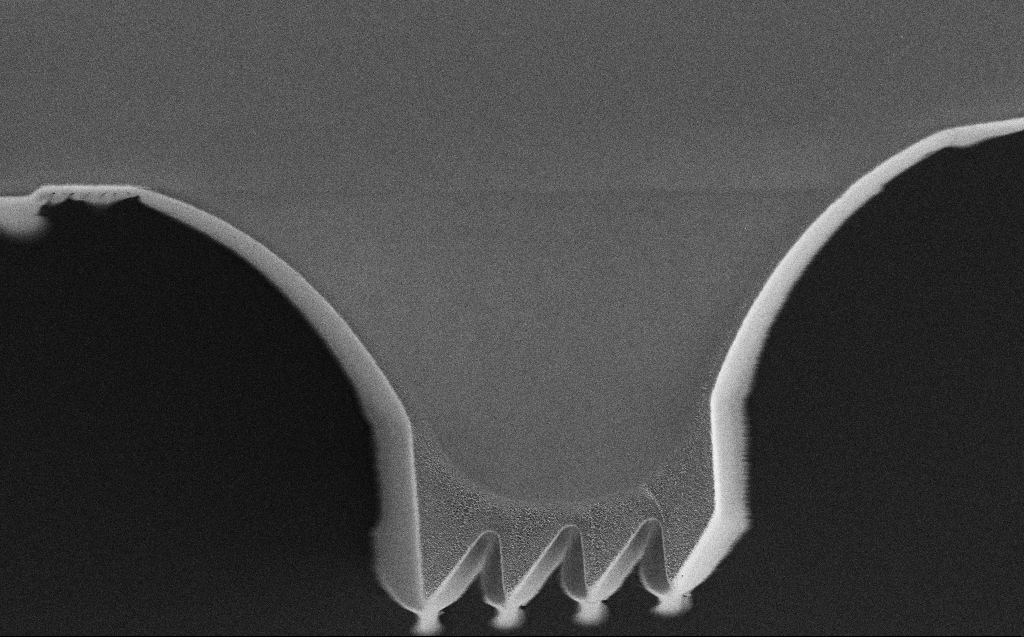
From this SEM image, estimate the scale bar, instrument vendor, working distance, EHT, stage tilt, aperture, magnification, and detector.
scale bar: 20000 nm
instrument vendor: Zeiss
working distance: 6 mm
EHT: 1 kV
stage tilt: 0°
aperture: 30 µm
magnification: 2.63 K X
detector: SE2